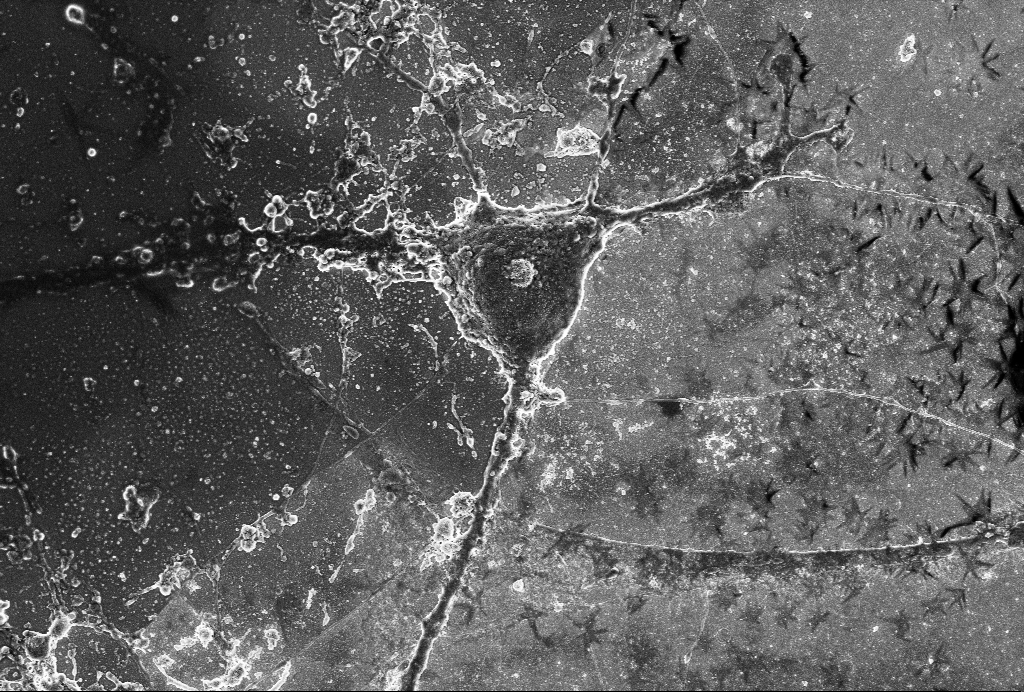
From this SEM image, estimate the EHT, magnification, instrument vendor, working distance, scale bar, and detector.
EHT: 4 kV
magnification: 4 K X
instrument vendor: Zeiss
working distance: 6 mm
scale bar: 10000 nm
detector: SE2